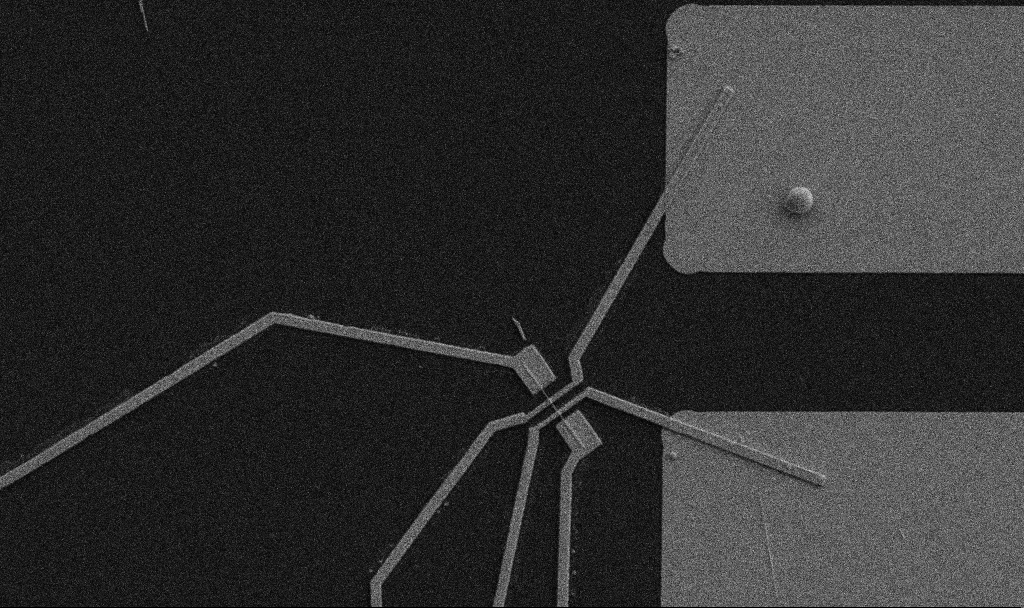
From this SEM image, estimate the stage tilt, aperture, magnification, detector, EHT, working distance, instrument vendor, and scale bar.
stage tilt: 0°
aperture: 30 µm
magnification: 5 K X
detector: SE2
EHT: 5 kV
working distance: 10.7 mm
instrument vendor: Zeiss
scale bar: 10000 nm